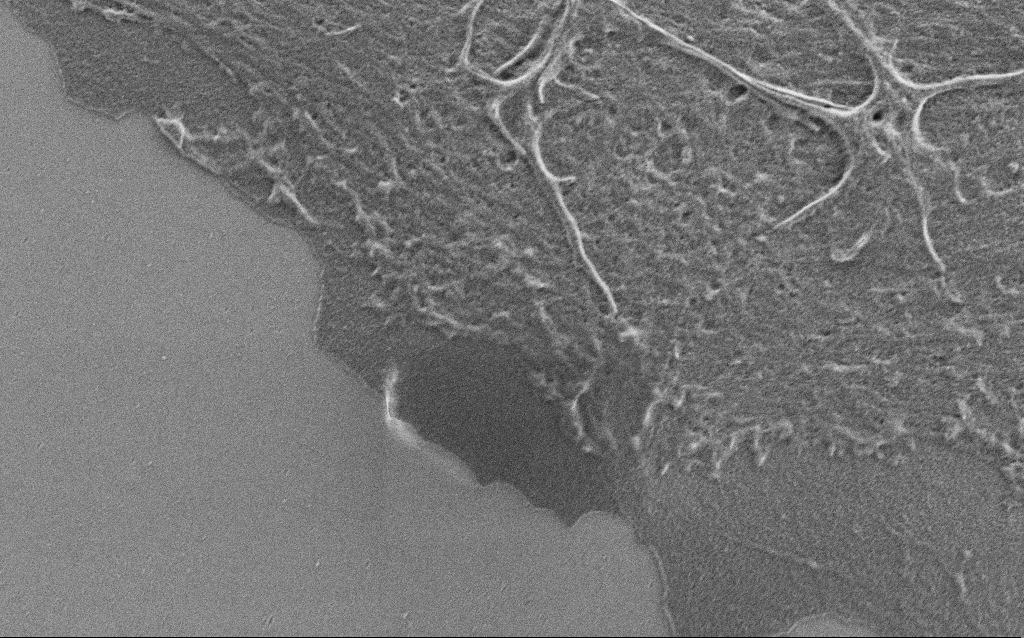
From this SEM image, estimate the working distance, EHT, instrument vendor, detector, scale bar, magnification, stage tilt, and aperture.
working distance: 4 mm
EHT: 0.9 kV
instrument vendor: Zeiss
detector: SE2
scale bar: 2000 nm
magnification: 15 K X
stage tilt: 0°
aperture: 30 µm